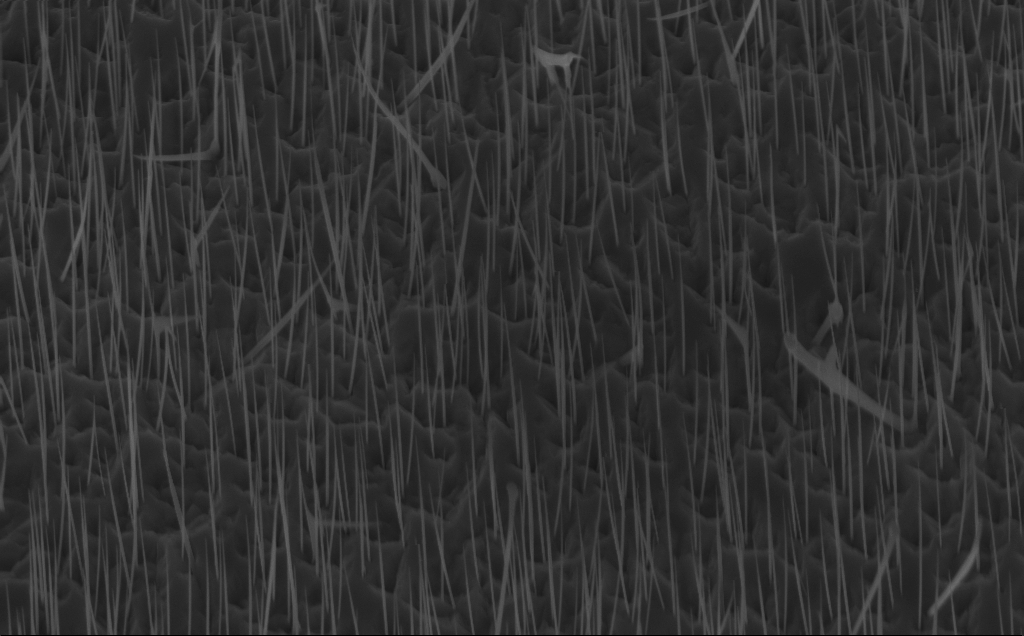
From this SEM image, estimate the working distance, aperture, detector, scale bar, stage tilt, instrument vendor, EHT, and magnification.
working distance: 7 mm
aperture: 30 µm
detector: InLens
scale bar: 1000 nm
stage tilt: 45°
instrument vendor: Zeiss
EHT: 10 kV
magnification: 20 K X